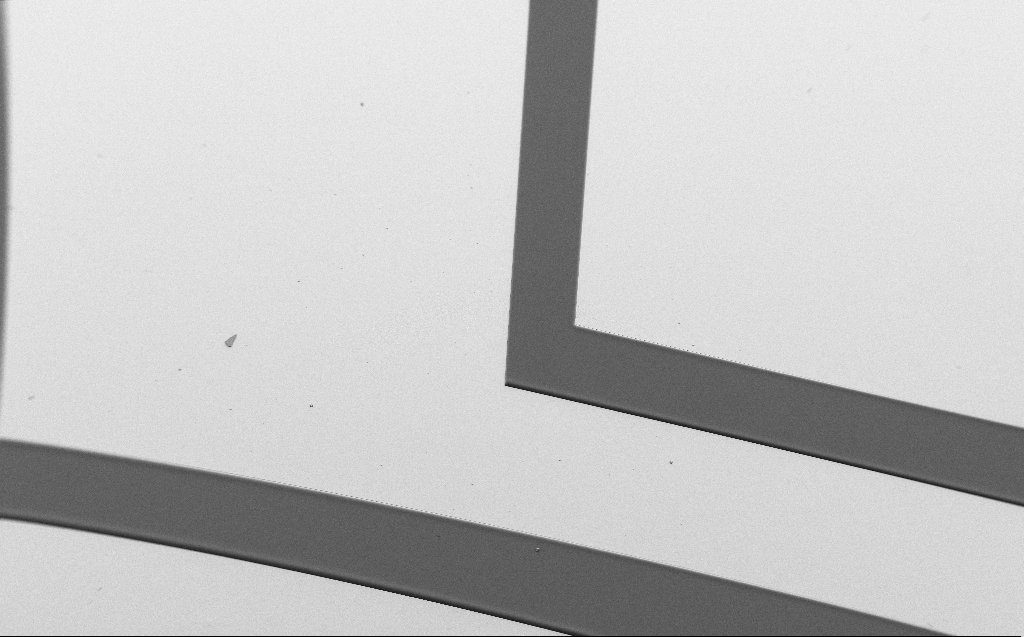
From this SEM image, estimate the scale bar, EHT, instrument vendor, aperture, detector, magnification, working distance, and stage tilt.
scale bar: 100000 nm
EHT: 1.1 kV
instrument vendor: Zeiss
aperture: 30 µm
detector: SE2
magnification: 0.25 K X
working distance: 6 mm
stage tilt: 30°